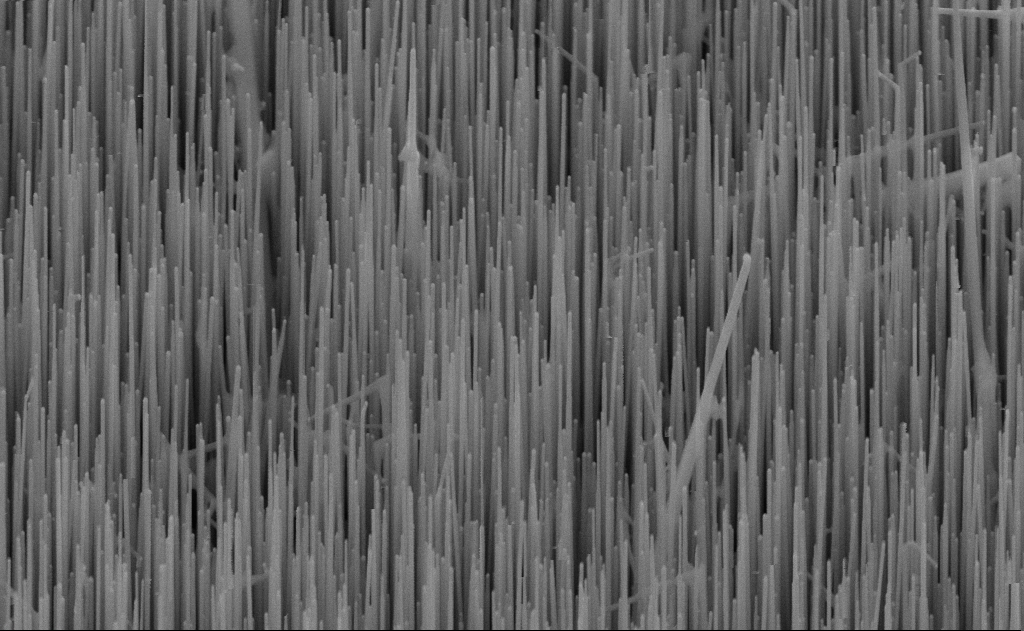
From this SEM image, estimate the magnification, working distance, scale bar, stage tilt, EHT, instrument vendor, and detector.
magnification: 40 K X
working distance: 10 mm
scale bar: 1000 nm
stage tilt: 45°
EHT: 10 kV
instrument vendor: Zeiss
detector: SE2